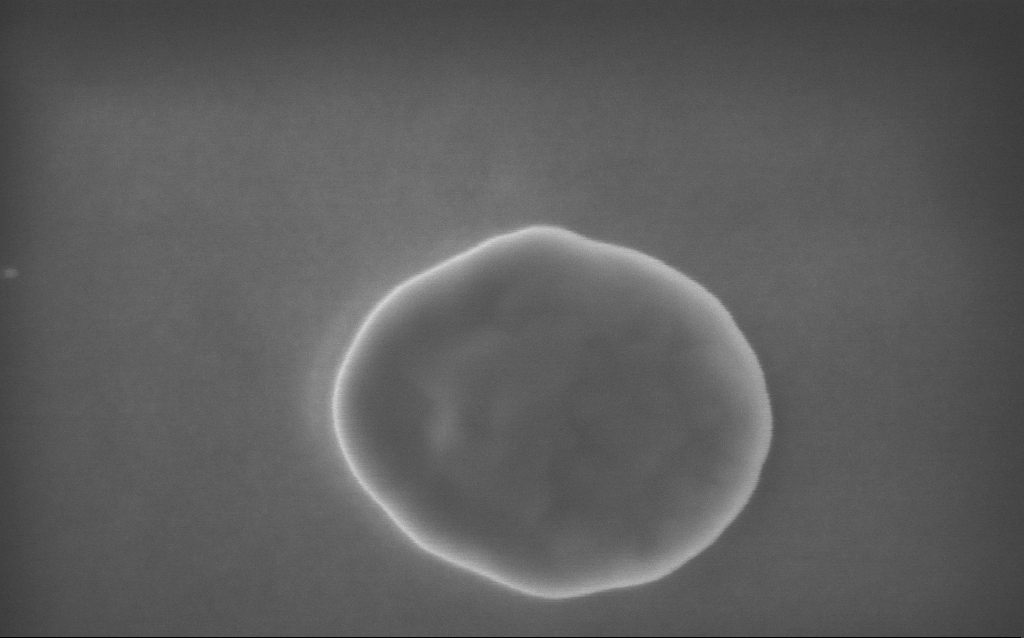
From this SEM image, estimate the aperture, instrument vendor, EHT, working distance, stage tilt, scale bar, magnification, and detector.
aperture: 30 µm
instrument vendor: Zeiss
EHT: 5 kV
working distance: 3 mm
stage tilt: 0°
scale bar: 200 nm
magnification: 175.17 K X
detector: InLens